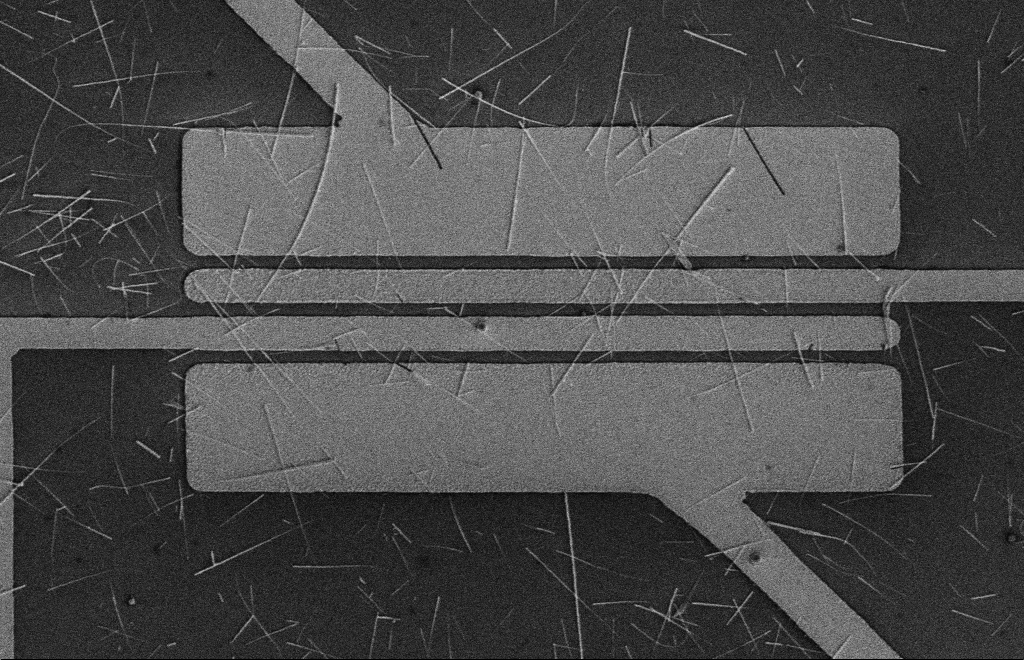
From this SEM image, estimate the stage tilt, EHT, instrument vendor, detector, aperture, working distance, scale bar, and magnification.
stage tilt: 0°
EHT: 5 kV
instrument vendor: Zeiss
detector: SE2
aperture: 10 µm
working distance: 16 mm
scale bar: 10000 nm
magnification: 4.34 K X